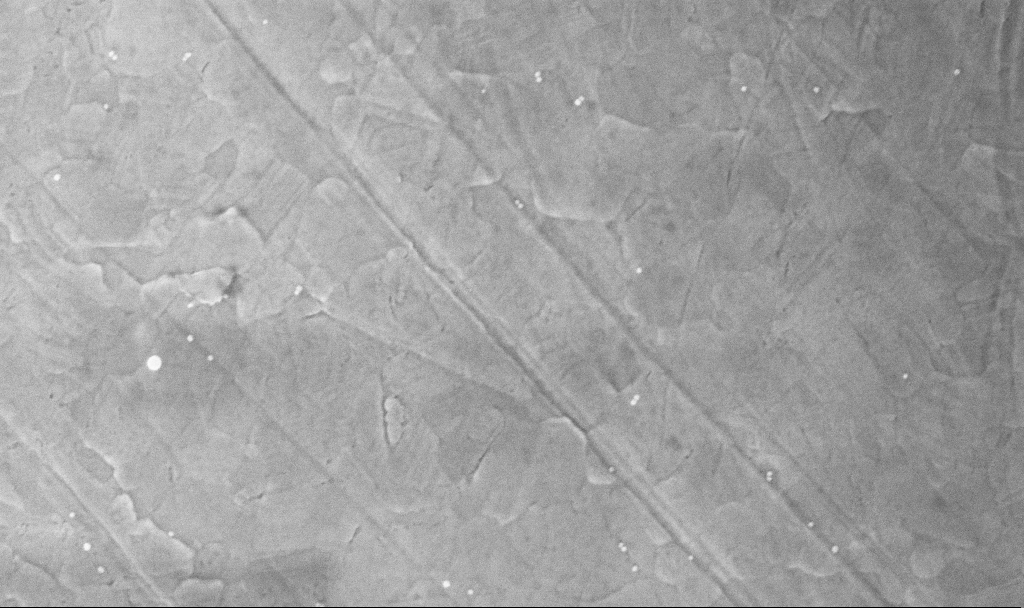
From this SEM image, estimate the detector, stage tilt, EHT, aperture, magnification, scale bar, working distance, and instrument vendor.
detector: InLens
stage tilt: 0°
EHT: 5 kV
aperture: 30 µm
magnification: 110.19 K X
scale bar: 200 nm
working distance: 3.7 mm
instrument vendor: Zeiss